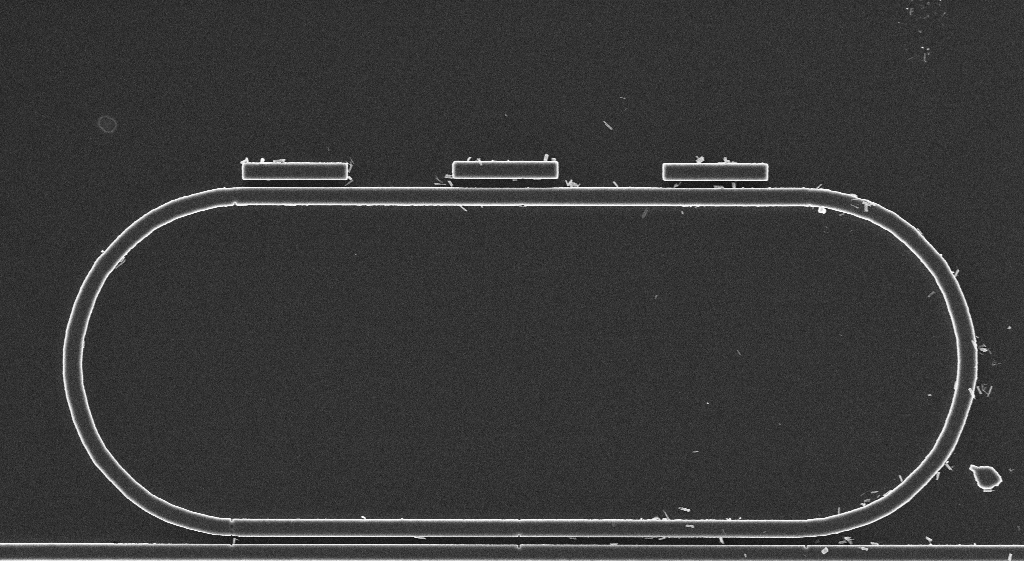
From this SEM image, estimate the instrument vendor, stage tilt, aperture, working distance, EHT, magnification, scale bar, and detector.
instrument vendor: Zeiss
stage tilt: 0°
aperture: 30 µm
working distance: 2.9 mm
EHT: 3 kV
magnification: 13.06 K X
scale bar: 2000 nm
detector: InLens